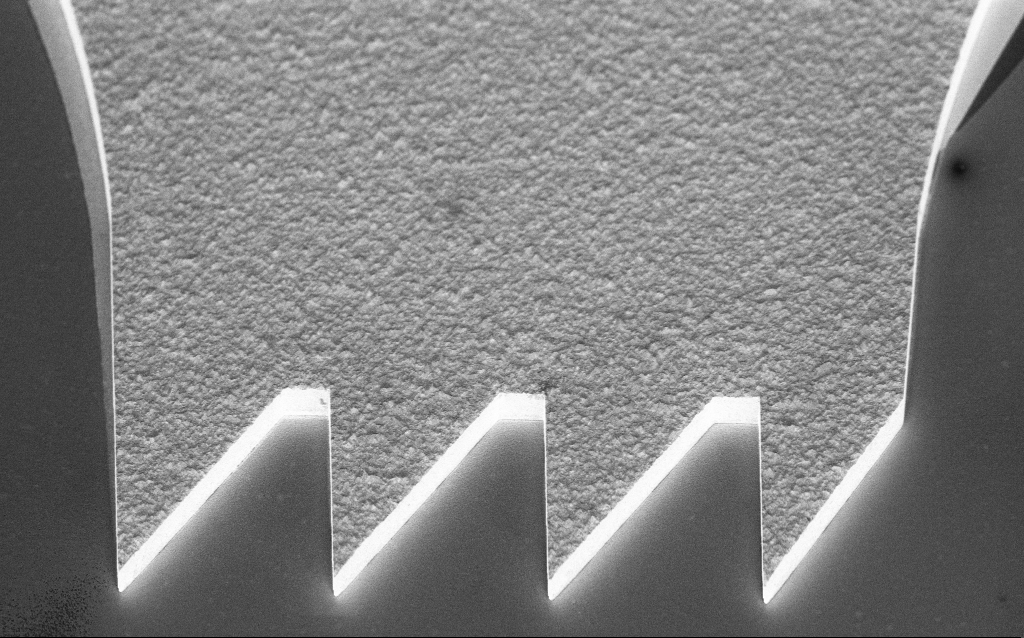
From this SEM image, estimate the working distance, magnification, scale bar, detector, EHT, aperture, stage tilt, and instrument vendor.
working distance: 6.4 mm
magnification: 6.97 K X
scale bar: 10000 nm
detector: InLens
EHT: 3 kV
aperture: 30 µm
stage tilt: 45°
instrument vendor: Zeiss